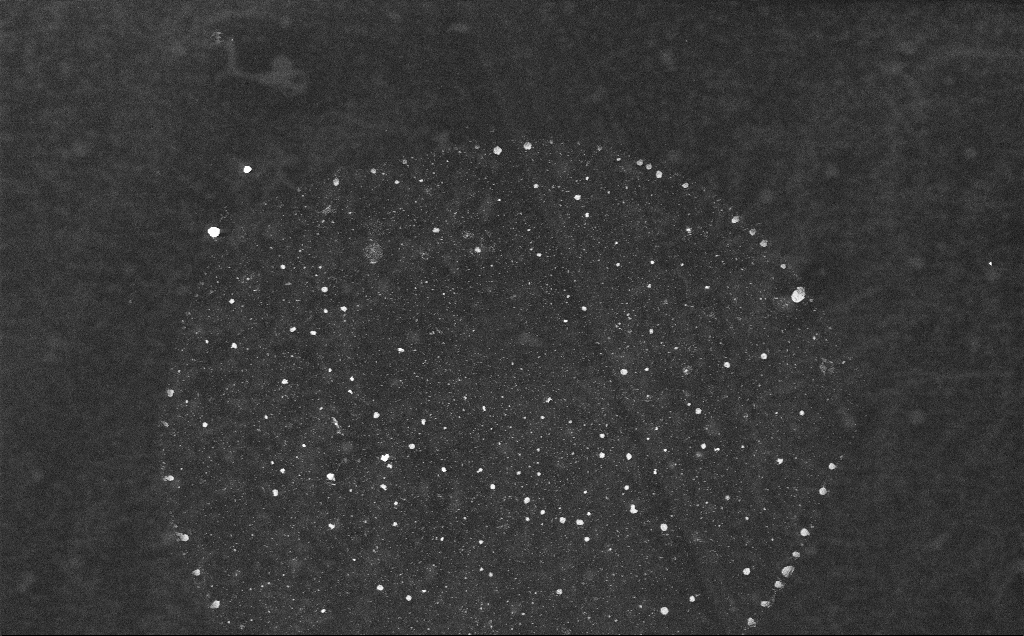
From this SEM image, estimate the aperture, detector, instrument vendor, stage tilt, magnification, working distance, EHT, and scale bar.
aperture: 30 µm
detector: InLens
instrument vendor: Zeiss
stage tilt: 0°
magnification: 2.34 K X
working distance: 3.7 mm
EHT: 10 kV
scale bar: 10000 nm